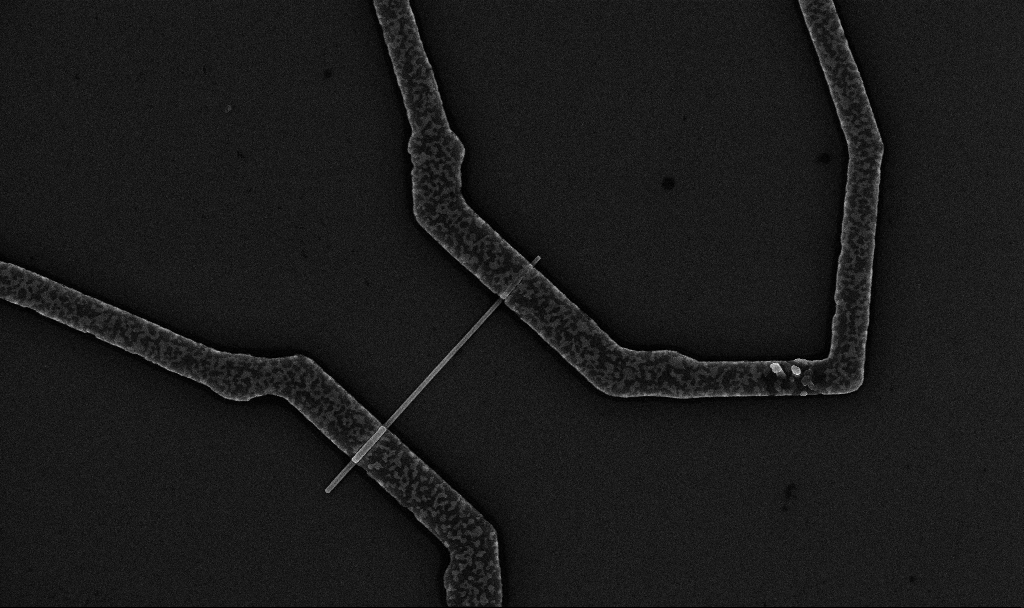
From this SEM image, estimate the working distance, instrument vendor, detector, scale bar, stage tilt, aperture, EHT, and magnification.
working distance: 6.7 mm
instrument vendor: Zeiss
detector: InLens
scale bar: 2000 nm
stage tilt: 0°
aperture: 30 µm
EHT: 10 kV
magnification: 17.95 K X